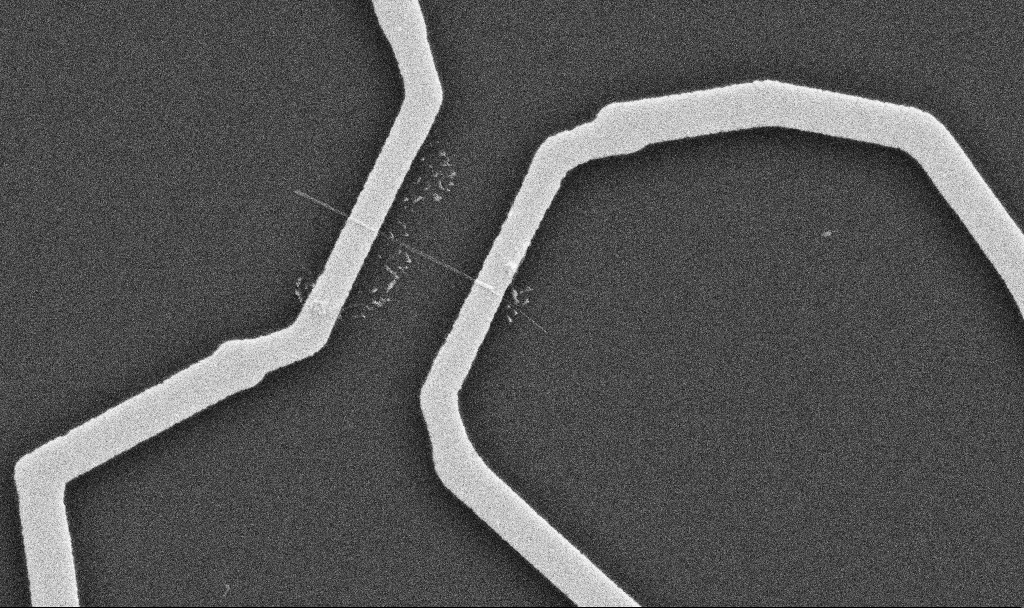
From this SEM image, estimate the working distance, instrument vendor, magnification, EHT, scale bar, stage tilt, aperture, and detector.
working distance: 10.7 mm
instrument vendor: Zeiss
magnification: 20 K X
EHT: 10 kV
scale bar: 1000 nm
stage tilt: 0°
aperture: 30 µm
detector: SE2